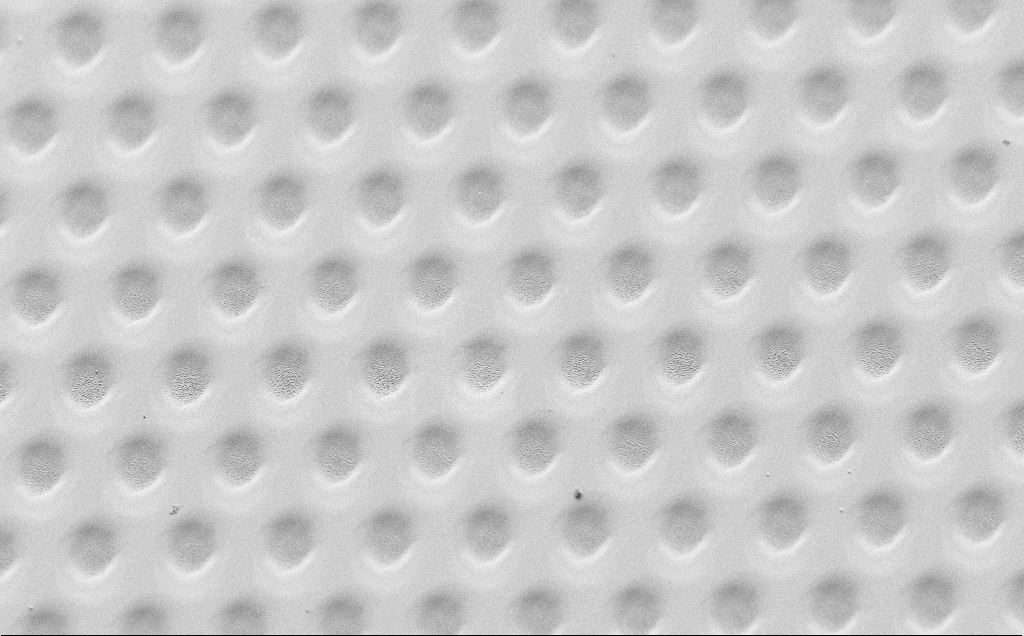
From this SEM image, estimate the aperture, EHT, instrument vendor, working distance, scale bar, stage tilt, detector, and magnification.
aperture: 30 µm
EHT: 1.5 kV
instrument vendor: Zeiss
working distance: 4 mm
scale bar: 10000 nm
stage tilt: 45°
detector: SE2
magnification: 1.85 K X